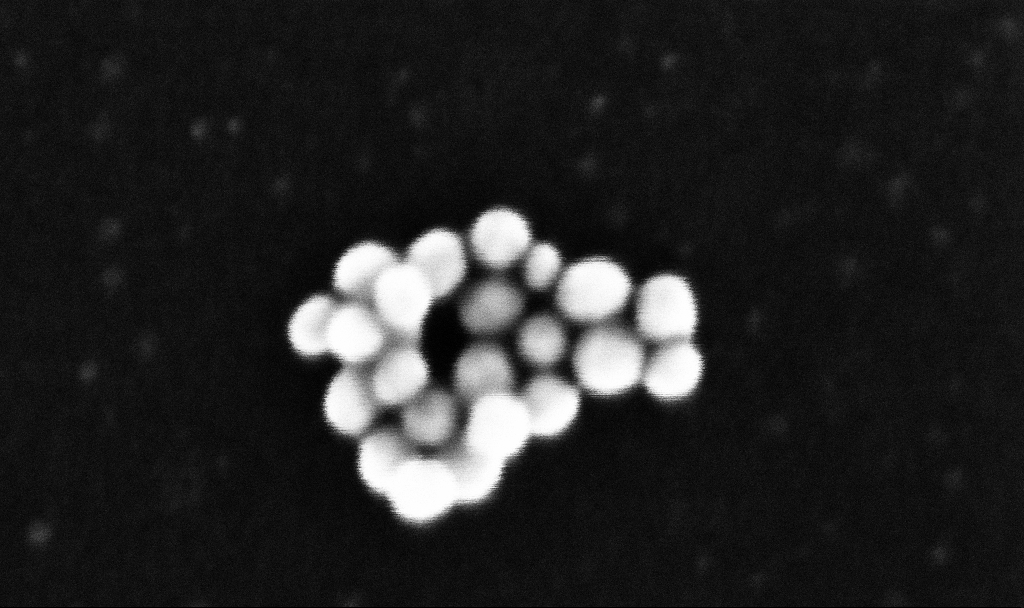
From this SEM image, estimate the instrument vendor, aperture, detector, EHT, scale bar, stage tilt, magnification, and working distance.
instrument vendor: Zeiss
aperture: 30 µm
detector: InLens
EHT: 10 kV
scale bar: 20 nm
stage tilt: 0°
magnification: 1100.44 K X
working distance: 3.2 mm